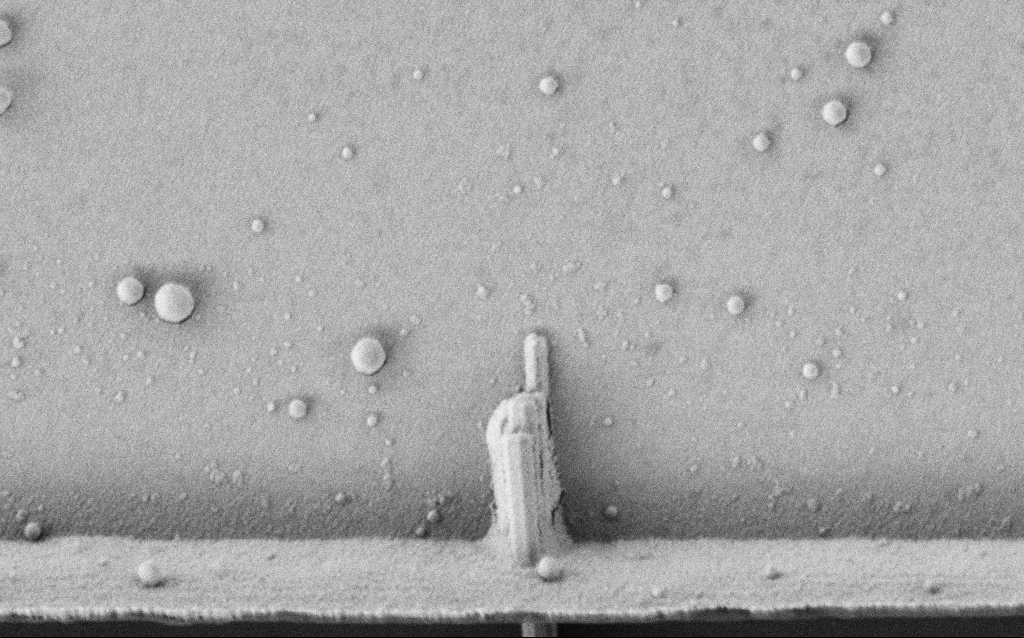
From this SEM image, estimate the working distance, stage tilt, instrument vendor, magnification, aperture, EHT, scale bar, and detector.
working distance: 7.7 mm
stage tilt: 0°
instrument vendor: Zeiss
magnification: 38.4 K X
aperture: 30 µm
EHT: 2 kV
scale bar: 1000 nm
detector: SE2